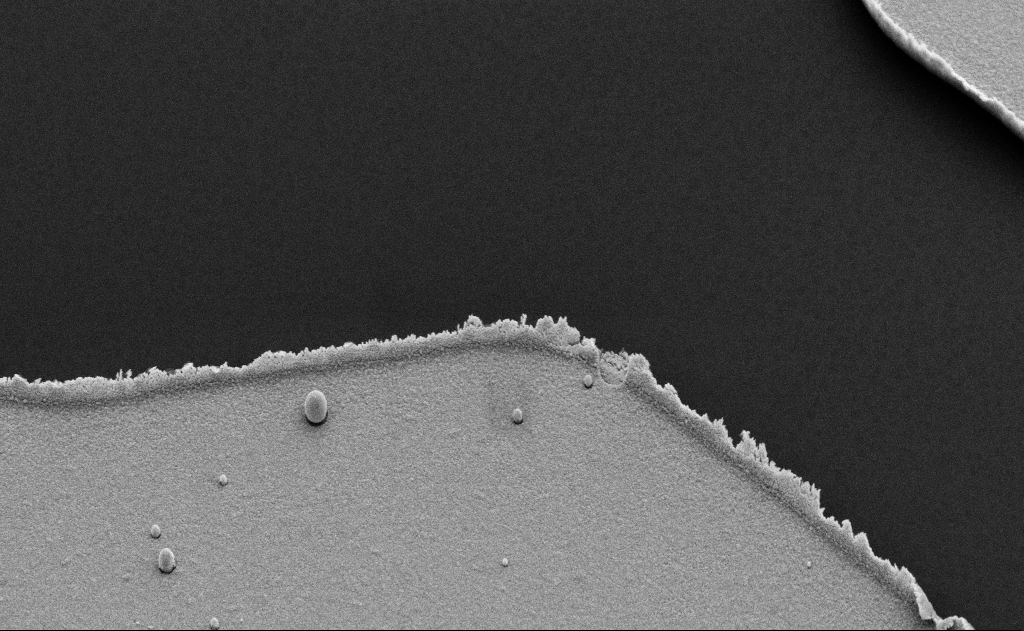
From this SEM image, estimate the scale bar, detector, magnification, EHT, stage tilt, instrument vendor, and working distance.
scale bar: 1000 nm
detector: SE2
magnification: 21.47 K X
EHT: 5 kV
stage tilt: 45°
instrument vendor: Zeiss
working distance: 10 mm